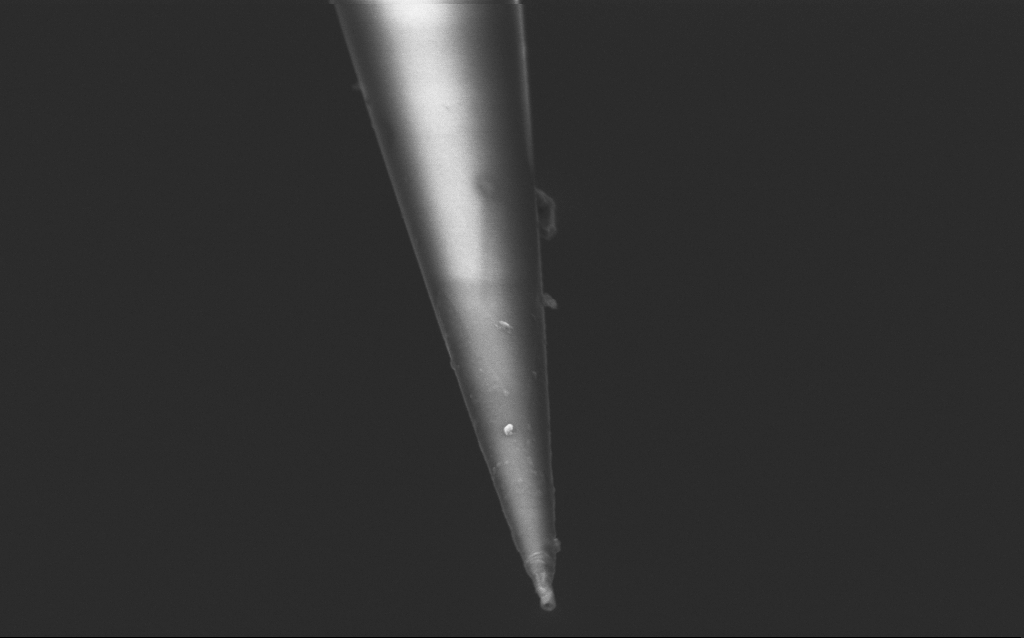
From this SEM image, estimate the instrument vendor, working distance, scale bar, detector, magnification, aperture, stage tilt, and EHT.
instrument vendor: Zeiss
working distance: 6 mm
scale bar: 1000 nm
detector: InLens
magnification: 50 K X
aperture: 30 µm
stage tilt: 45°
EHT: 2.5 kV